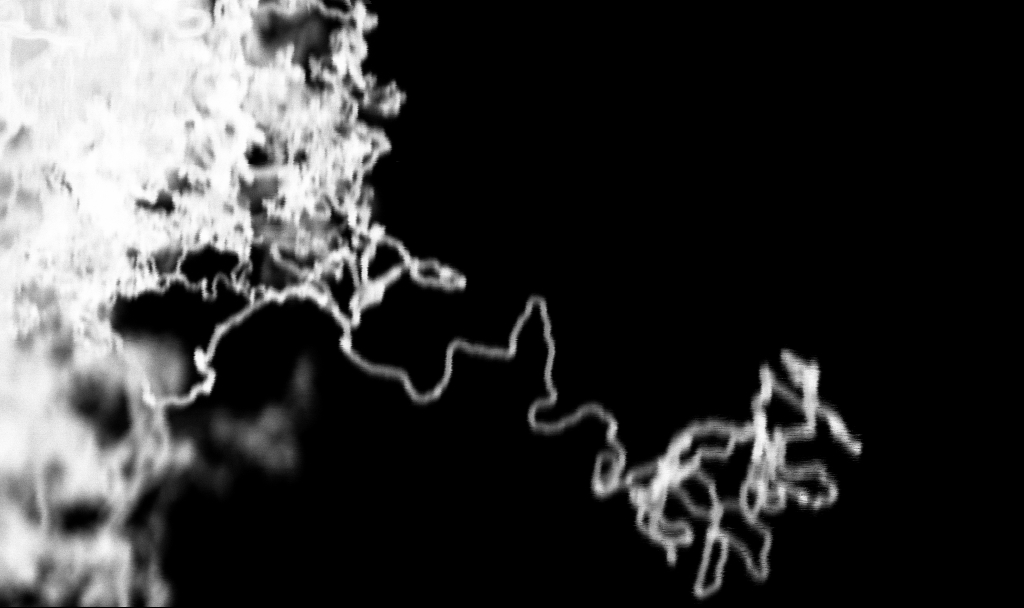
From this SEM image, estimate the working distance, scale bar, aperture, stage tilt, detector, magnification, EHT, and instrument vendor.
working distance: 2.7 mm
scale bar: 200 nm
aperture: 30 µm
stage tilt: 0°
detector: InLens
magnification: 100 K X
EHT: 3 kV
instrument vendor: Zeiss